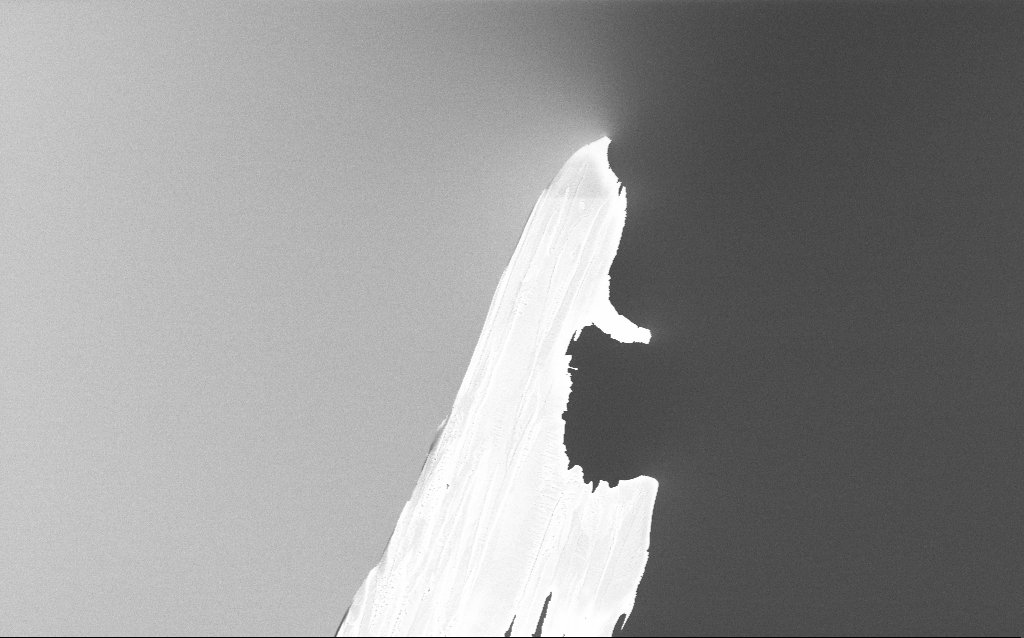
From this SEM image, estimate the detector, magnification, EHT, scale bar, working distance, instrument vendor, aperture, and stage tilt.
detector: InLens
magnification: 16.21 K X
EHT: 5 kV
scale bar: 1000 nm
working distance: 2.9 mm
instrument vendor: Zeiss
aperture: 30 µm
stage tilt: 0°